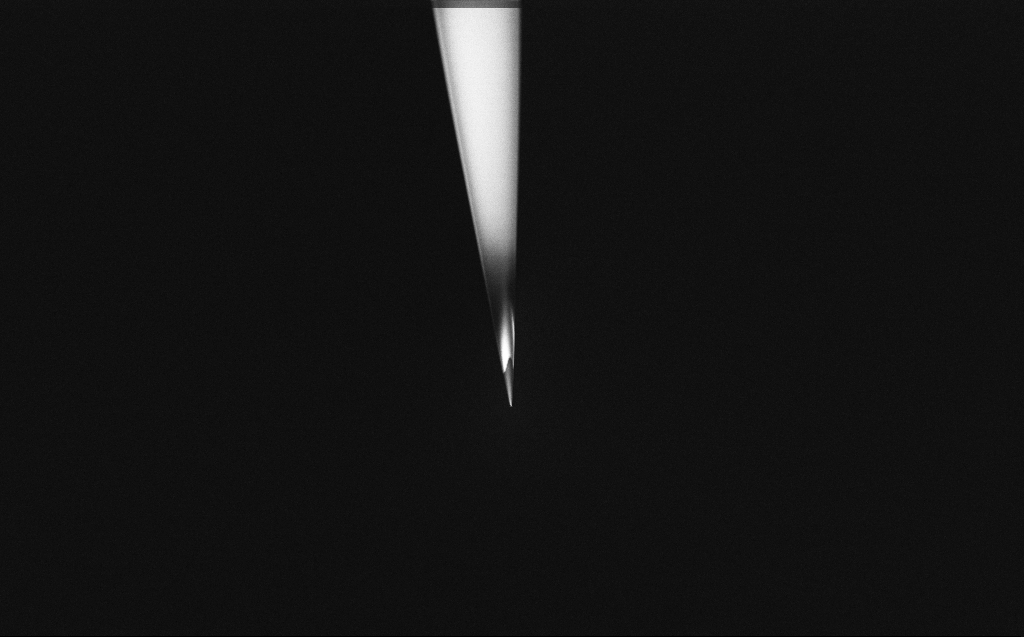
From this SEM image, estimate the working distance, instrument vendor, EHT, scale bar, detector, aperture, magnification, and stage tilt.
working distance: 6 mm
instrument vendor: Zeiss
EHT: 2 kV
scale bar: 20000 nm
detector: InLens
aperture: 30 µm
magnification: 2.5 K X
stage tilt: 45°